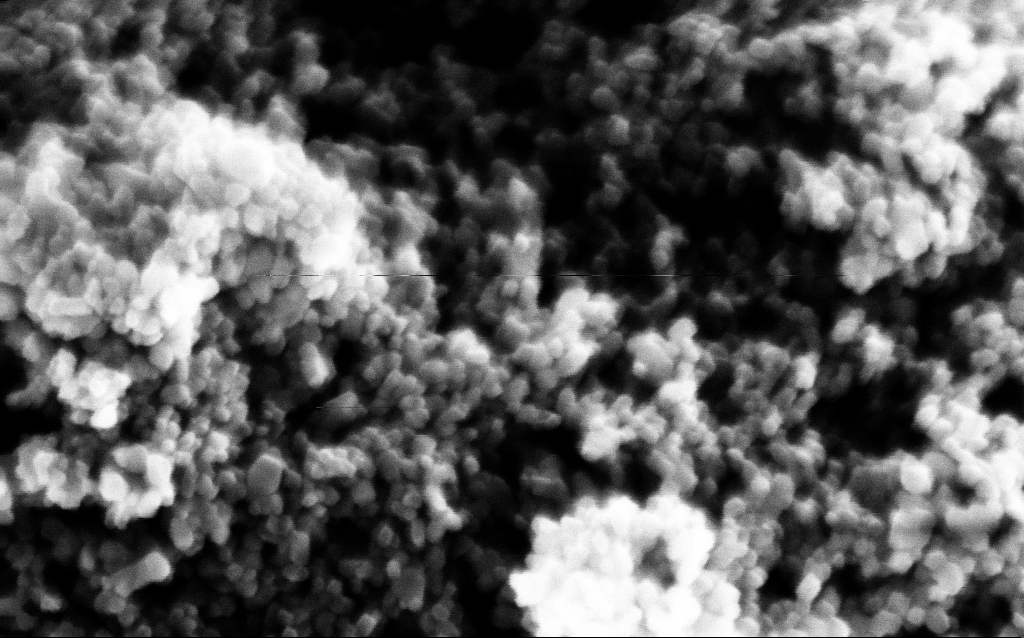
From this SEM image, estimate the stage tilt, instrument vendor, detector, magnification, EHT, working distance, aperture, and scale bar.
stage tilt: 0°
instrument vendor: Zeiss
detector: InLens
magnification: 286.5 K X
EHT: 5 kV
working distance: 1.8 mm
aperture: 30 µm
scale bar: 200 nm